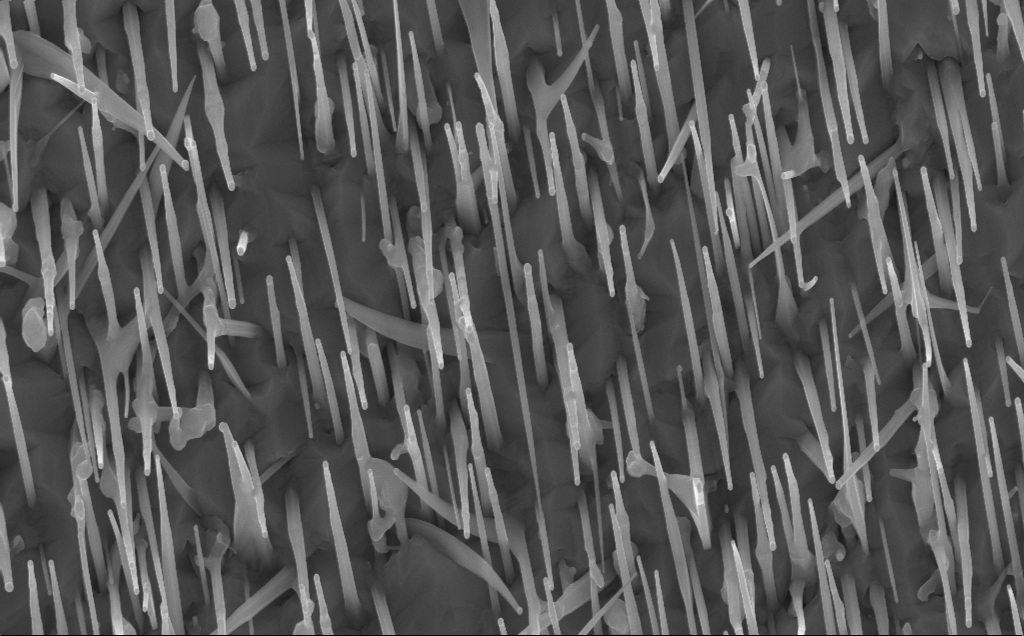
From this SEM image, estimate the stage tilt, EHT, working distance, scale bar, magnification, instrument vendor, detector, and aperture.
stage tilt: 0°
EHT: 10 kV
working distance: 7 mm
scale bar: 1000 nm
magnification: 40 K X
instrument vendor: Zeiss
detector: InLens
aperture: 30 µm